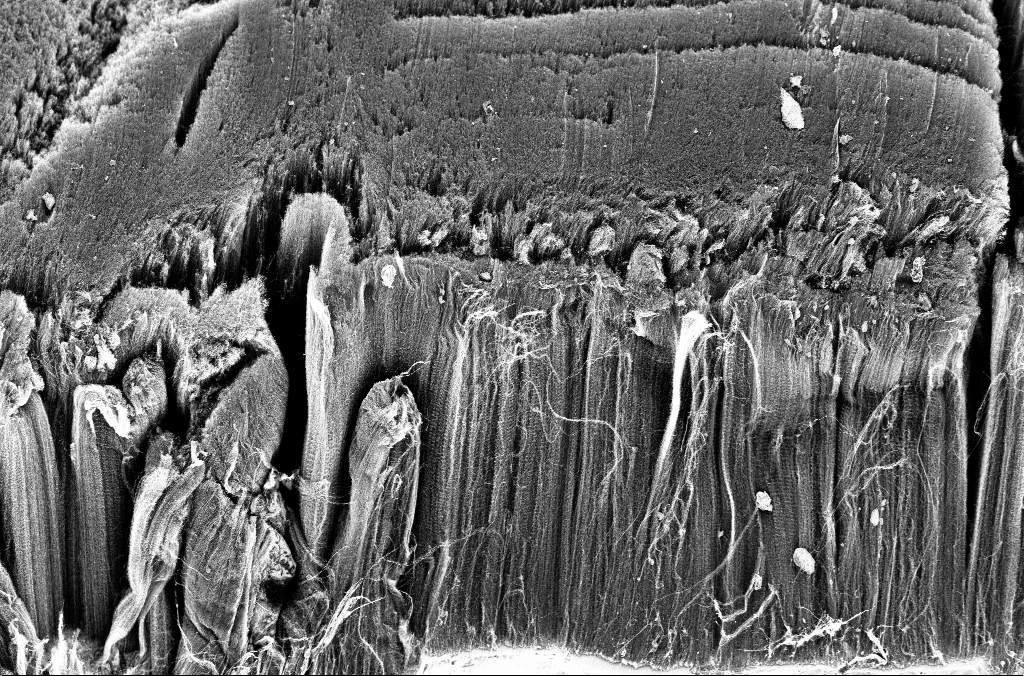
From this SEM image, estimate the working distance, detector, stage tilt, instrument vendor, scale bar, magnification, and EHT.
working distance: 3.3 mm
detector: InLens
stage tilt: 45°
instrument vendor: Zeiss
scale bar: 10000 nm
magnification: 1.25 K X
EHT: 3 kV